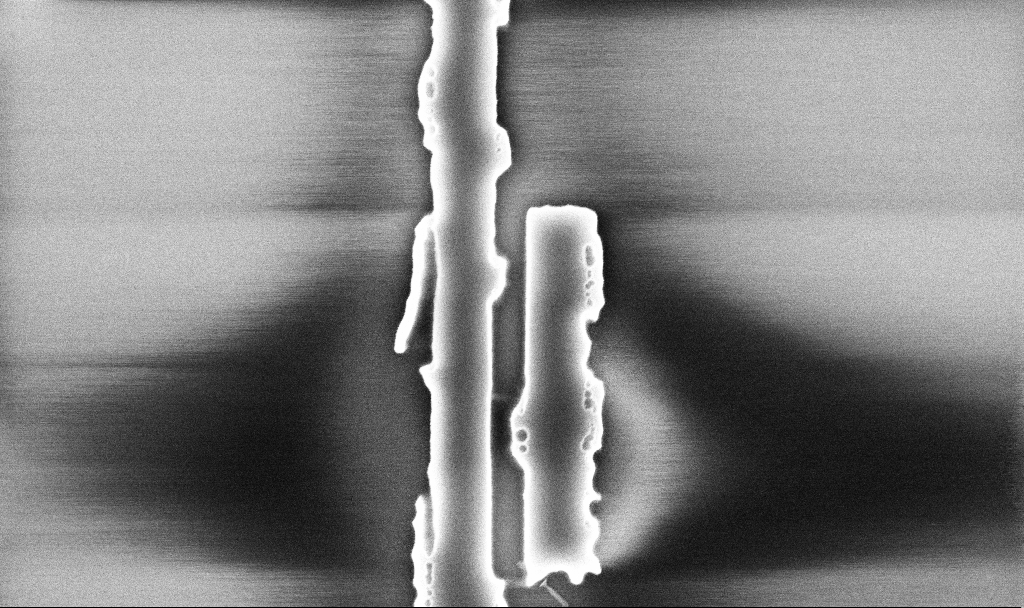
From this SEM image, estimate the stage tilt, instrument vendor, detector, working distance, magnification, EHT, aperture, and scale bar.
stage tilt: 0°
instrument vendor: Zeiss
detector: InLens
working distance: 10.1 mm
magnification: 45.07 K X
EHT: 5 kV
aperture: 30 µm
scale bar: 1000 nm